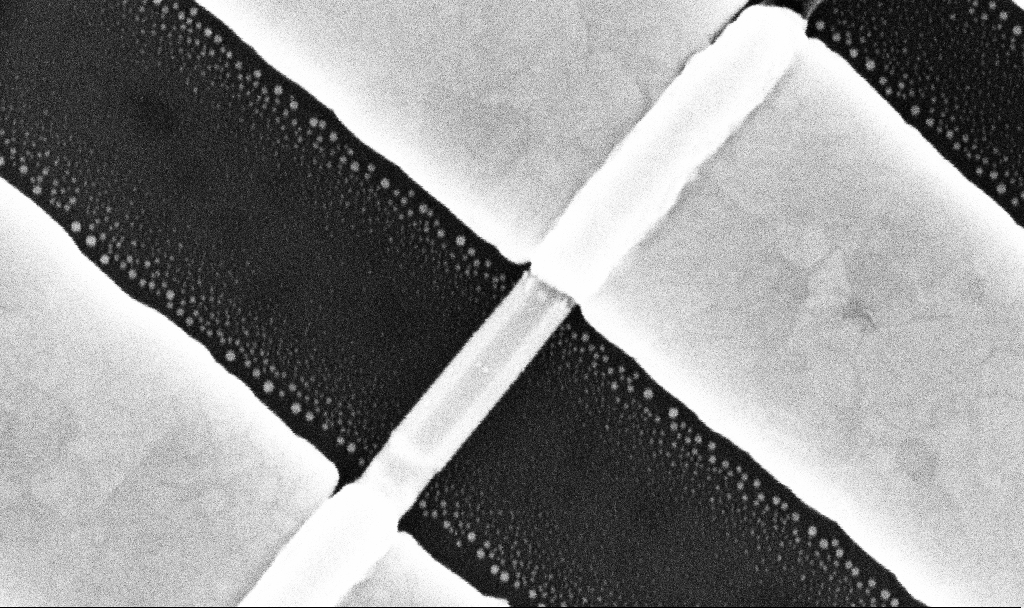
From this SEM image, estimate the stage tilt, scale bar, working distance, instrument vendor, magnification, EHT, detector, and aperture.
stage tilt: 0°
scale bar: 200 nm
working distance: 7.7 mm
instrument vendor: Zeiss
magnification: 203.38 K X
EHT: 10 kV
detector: InLens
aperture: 30 µm